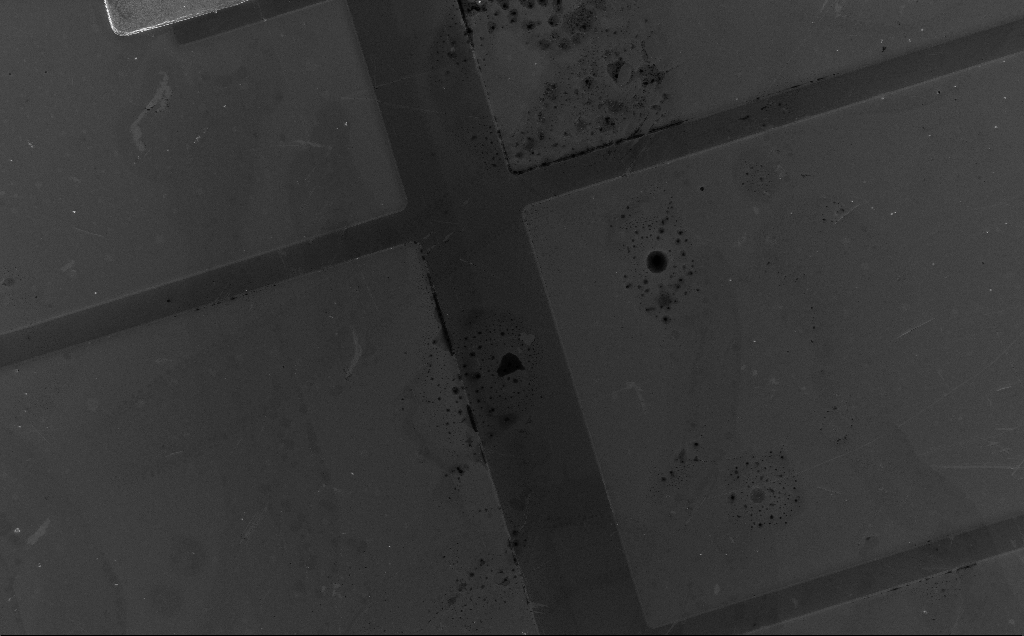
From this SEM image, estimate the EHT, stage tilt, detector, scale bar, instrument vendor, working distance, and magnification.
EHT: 5 kV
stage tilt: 0°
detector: InLens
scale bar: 100000 nm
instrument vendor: Zeiss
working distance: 6 mm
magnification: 0.676 K X